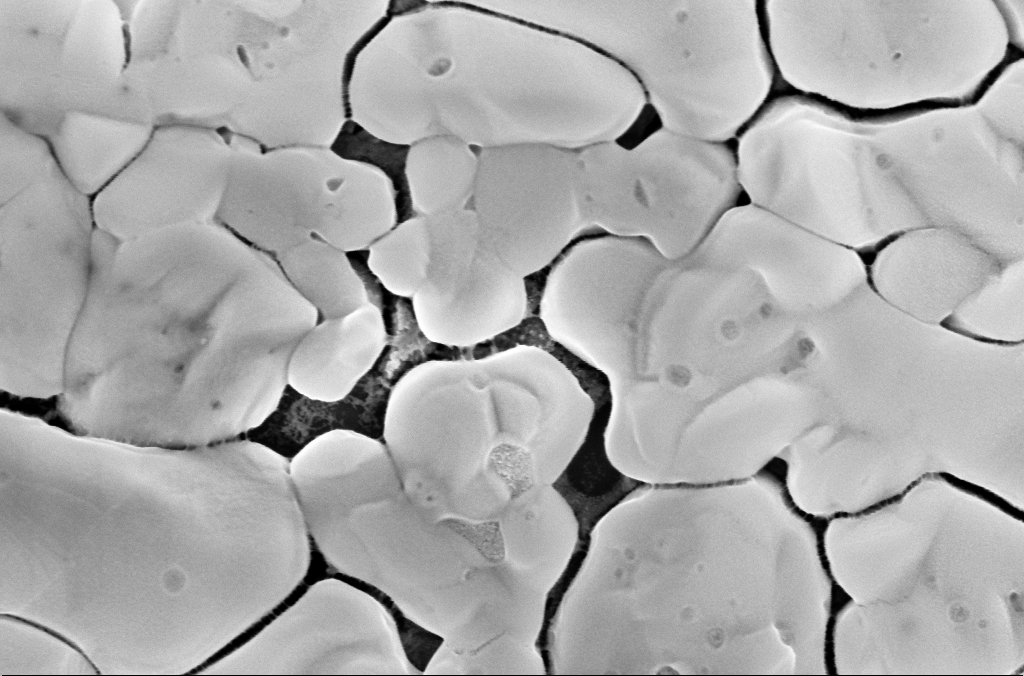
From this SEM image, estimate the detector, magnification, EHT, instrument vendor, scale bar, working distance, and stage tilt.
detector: InLens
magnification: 100 K X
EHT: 5 kV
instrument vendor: Zeiss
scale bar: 200 nm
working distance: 3.1 mm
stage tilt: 0°